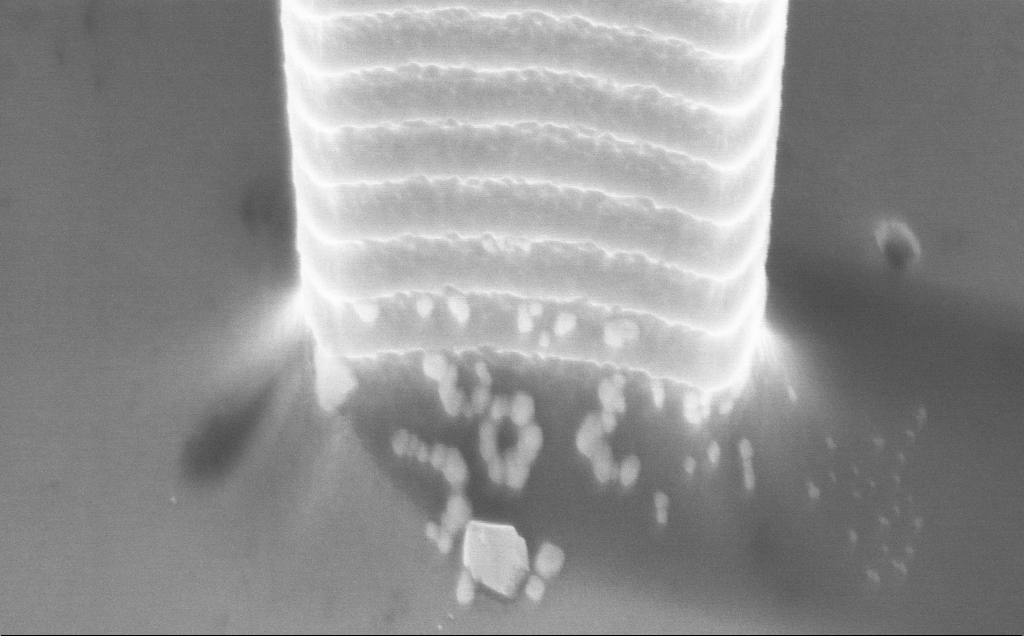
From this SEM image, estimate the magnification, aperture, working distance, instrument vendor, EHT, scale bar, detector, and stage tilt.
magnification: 53.13 K X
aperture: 30 µm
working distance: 5 mm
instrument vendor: Zeiss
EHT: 7.5 kV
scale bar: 1000 nm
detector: InLens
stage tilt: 45°